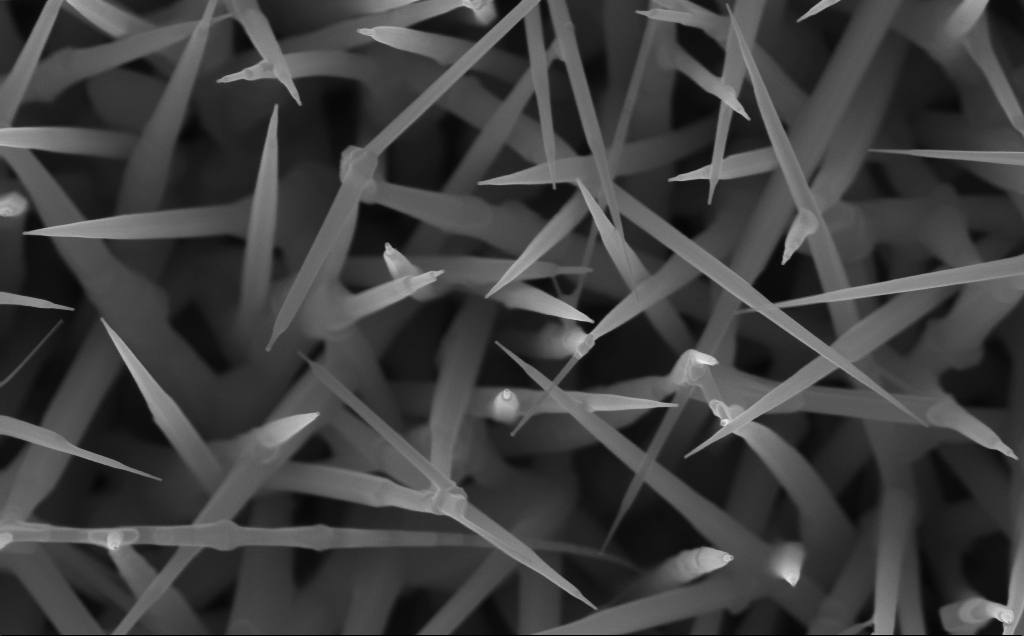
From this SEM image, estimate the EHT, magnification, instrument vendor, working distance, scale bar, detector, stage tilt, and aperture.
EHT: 10 kV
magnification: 80 K X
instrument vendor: Zeiss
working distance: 5 mm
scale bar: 200 nm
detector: InLens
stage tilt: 0°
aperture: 30 µm